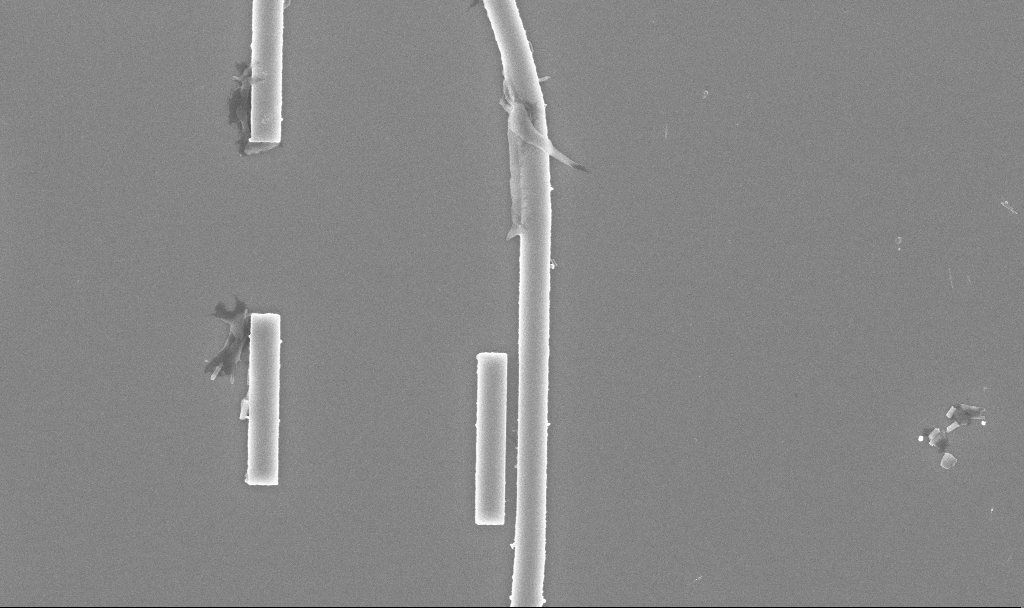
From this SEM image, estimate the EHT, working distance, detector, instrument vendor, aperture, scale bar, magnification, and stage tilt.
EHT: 5 kV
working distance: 9.9 mm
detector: InLens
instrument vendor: Zeiss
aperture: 30 µm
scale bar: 2000 nm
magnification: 21.1 K X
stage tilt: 0°